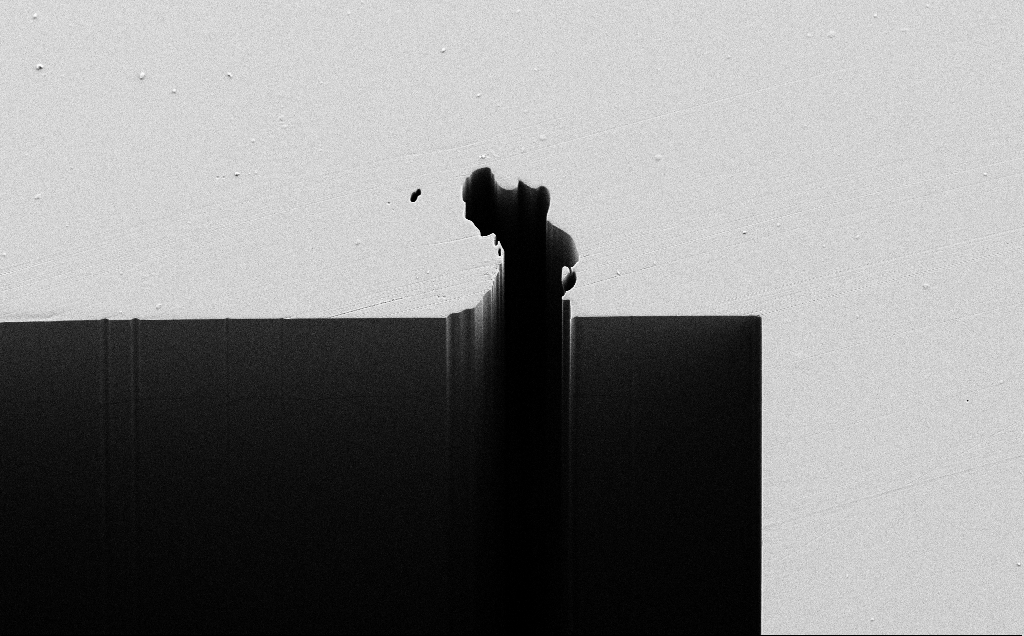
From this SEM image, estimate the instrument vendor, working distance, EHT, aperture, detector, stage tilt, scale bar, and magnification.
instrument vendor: Zeiss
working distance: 7 mm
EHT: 5 kV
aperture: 30 µm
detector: SE2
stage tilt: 30°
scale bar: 20000 nm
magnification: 0.724 K X